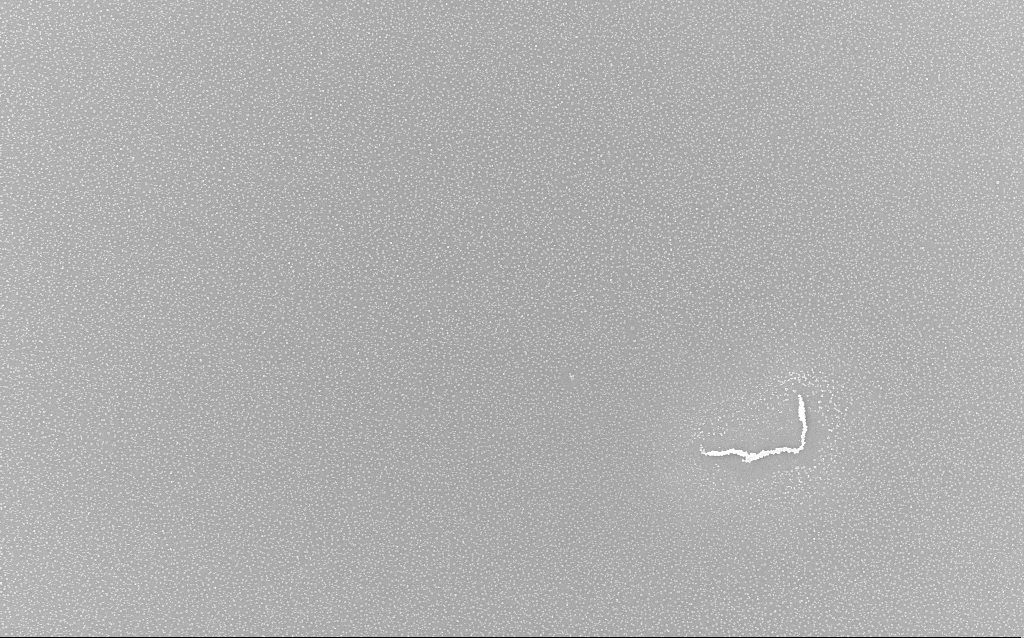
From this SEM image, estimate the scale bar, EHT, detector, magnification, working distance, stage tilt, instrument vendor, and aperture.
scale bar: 20000 nm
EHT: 20 kV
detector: InLens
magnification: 1 K X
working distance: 1.9 mm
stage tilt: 0°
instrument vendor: Zeiss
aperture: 30 µm